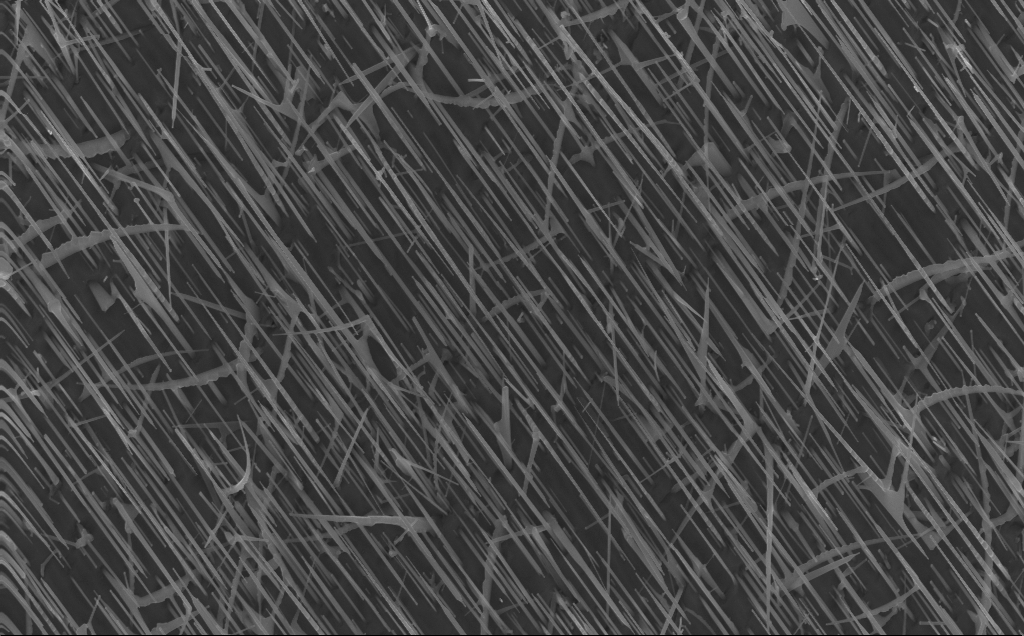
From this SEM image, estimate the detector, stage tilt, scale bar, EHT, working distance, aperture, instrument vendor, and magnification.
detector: InLens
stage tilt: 0°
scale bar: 2000 nm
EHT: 10 kV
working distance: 4 mm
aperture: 30 µm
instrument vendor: Zeiss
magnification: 20 K X